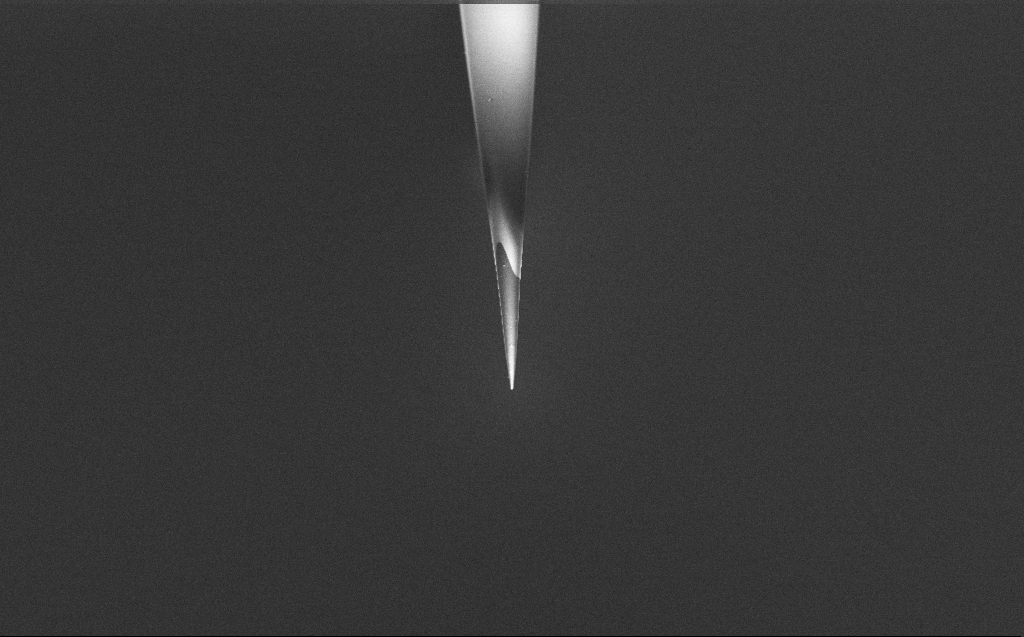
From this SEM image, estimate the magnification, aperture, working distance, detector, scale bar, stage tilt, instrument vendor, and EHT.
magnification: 2.5 K X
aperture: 30 µm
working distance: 6 mm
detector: InLens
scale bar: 20000 nm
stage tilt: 45°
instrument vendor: Zeiss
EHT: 2 kV